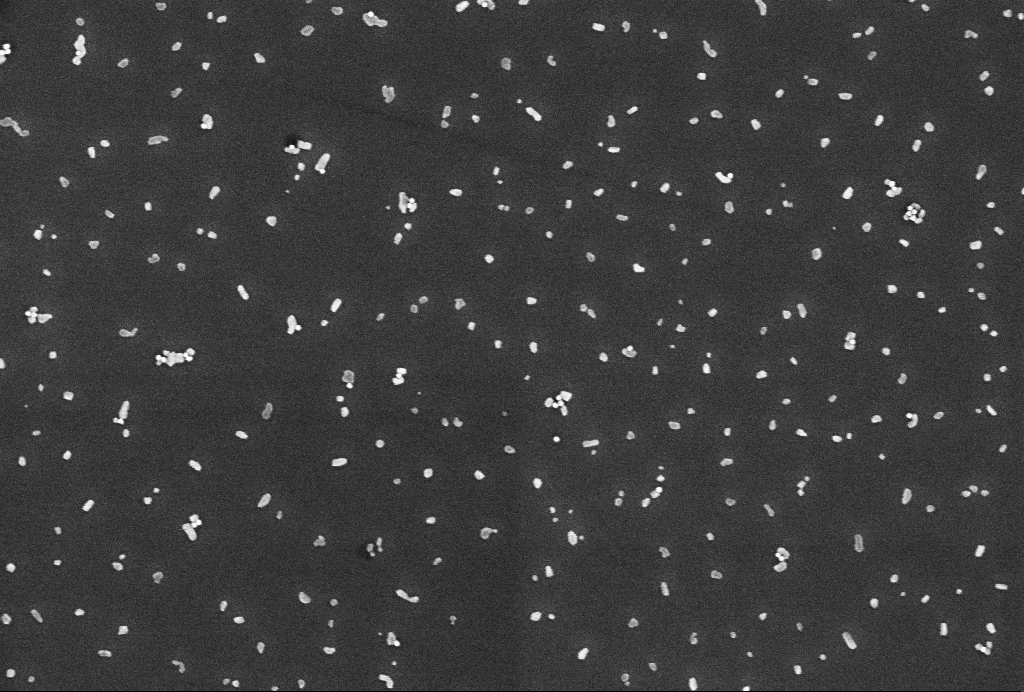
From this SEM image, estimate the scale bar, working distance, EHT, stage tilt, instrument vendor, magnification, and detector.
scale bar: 200 nm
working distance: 3.3 mm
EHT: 2 kV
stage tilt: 0°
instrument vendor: Zeiss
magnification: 86.18 K X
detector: InLens